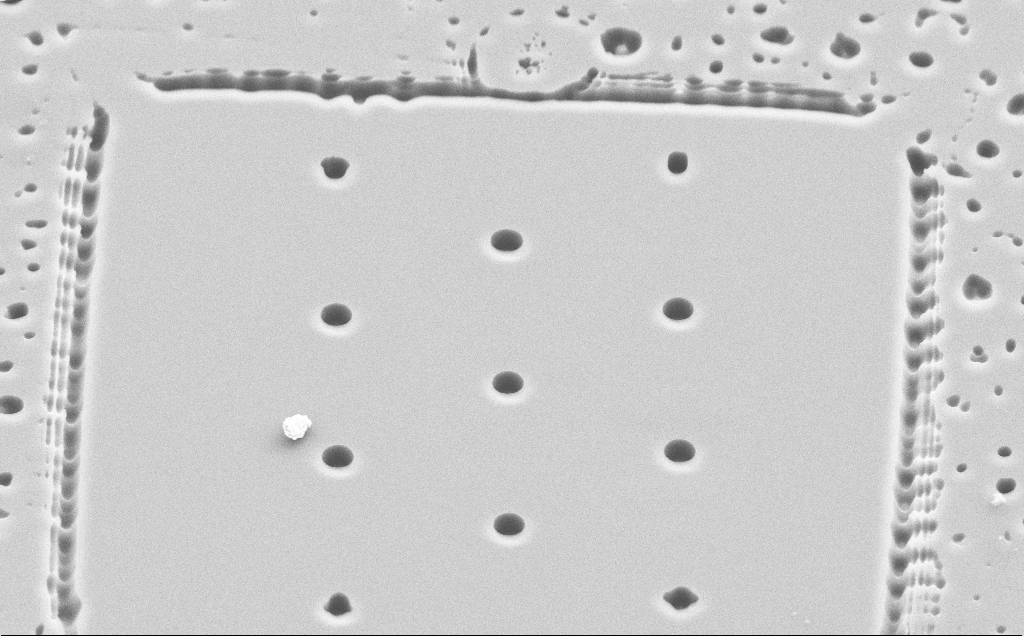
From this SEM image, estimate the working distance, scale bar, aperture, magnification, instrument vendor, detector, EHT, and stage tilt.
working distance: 6 mm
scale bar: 10000 nm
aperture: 30 µm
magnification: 3.56 K X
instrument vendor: Zeiss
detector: SE2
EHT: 10 kV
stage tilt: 45°